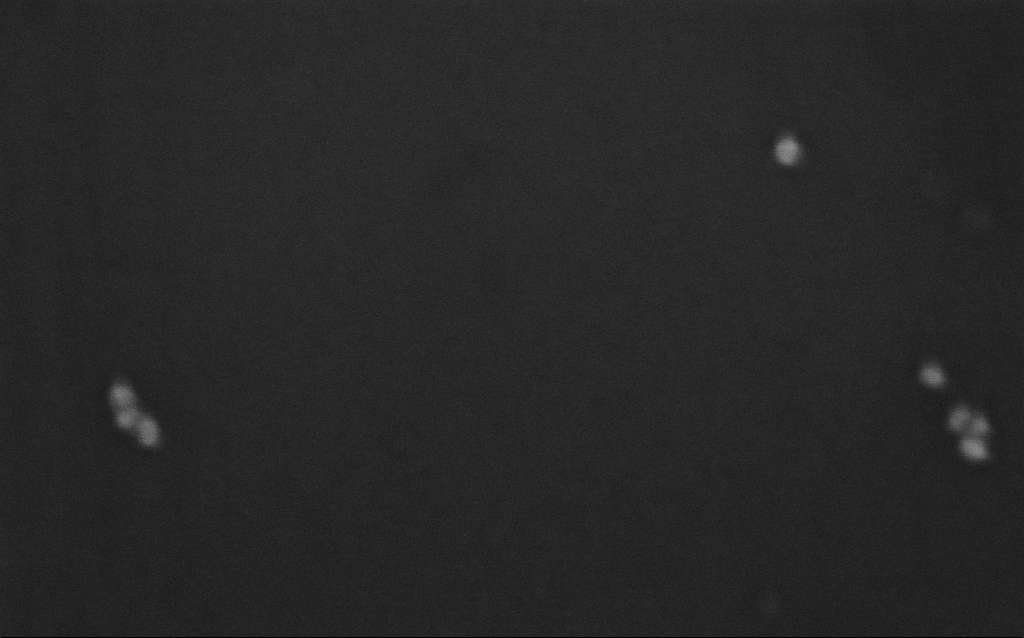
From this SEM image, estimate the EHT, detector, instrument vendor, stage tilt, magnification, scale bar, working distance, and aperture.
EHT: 10 kV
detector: InLens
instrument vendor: Zeiss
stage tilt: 0°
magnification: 500 K X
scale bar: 100 nm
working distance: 7 mm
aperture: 30 µm